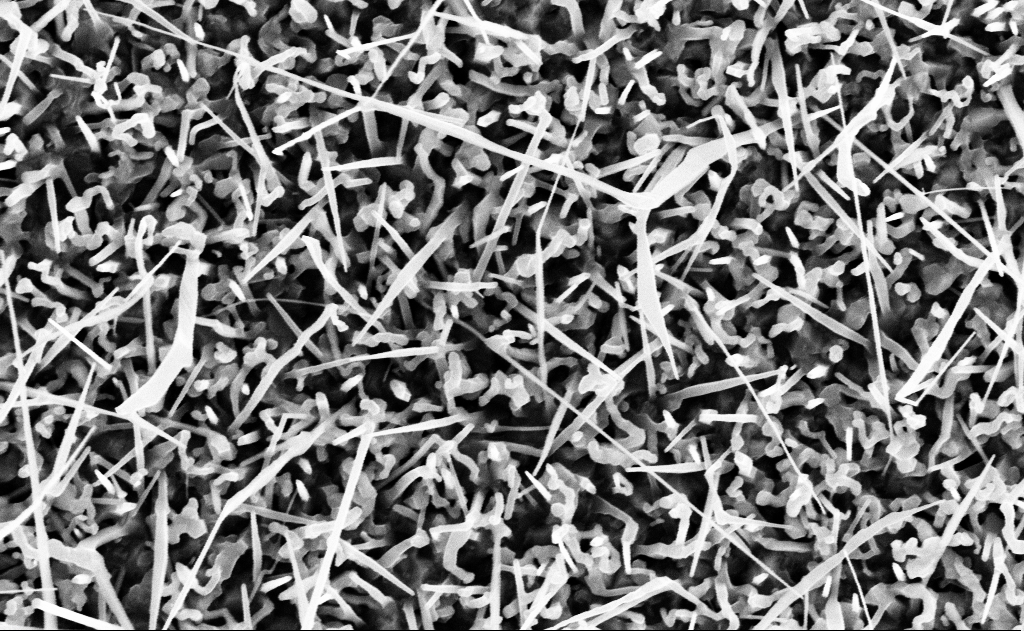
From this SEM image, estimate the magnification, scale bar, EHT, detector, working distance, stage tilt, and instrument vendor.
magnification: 40 K X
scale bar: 1000 nm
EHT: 10 kV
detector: InLens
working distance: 15 mm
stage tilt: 0°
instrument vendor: Zeiss